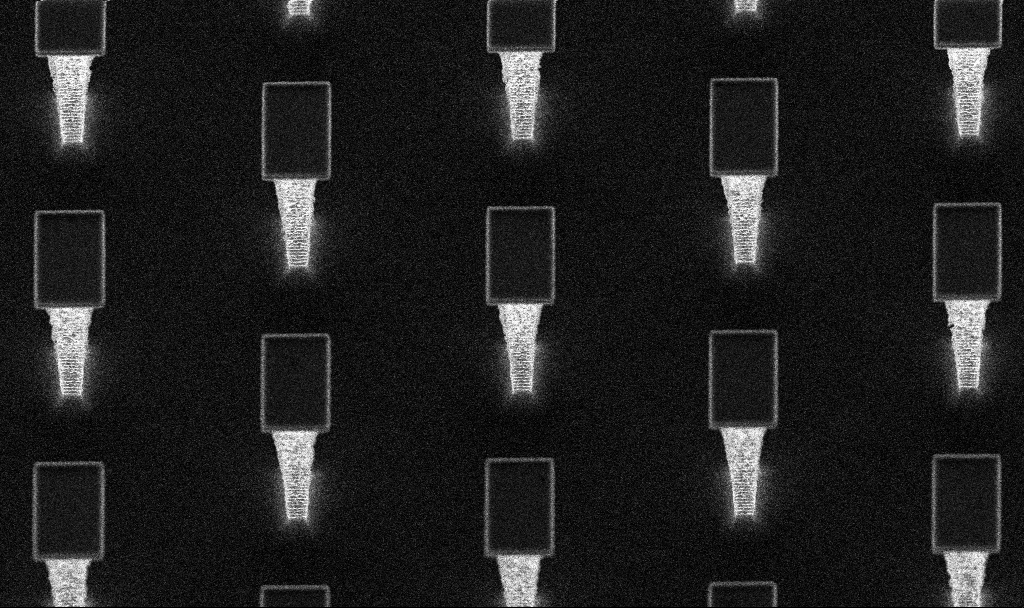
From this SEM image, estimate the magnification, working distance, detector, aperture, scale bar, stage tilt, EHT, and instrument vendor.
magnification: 8.26 K X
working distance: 4.2 mm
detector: InLens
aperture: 30 µm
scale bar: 2000 nm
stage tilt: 20°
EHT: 5 kV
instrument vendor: Zeiss